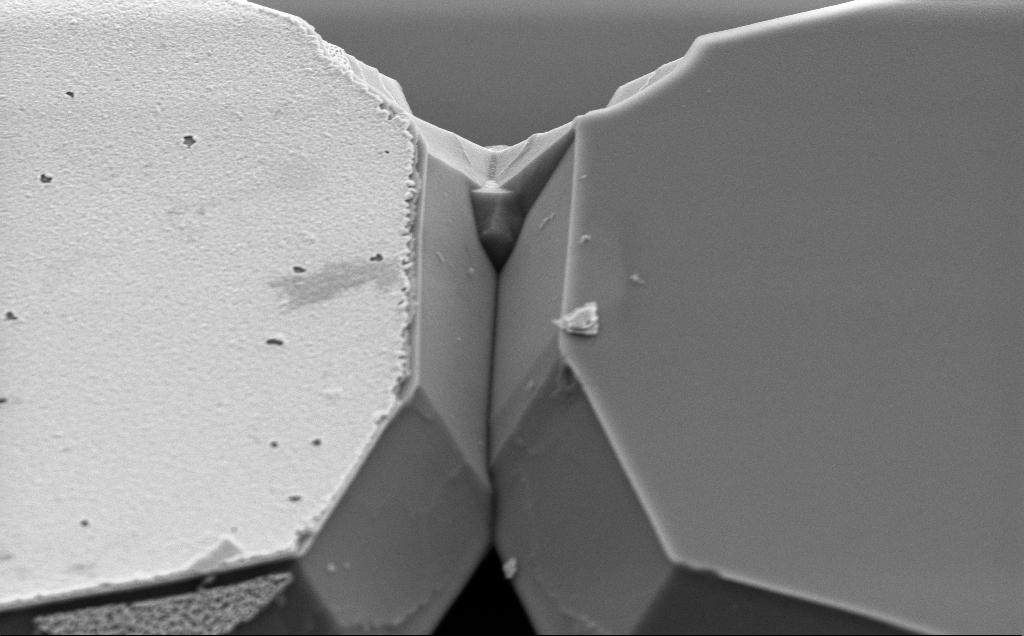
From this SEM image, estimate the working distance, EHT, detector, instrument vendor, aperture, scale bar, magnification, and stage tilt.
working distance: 10 mm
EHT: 5 kV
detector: SE2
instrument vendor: Zeiss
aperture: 30 µm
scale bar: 2000 nm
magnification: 21.32 K X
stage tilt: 50°